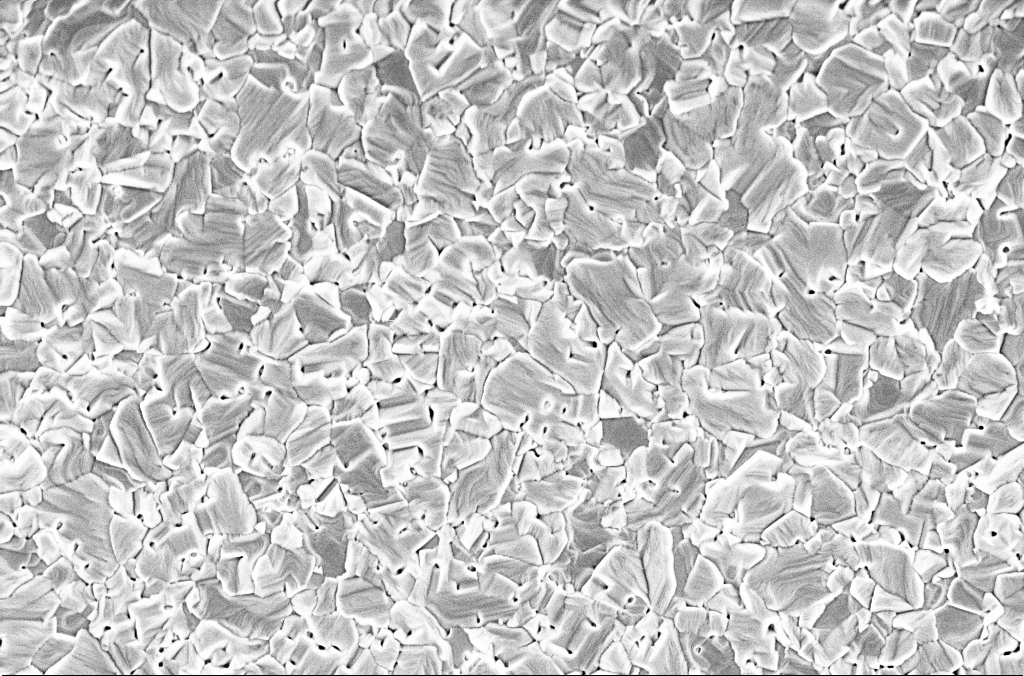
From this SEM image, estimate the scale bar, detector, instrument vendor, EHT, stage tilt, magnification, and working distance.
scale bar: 2000 nm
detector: InLens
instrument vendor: Zeiss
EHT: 2 kV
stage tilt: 0°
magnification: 30 K X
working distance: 3 mm